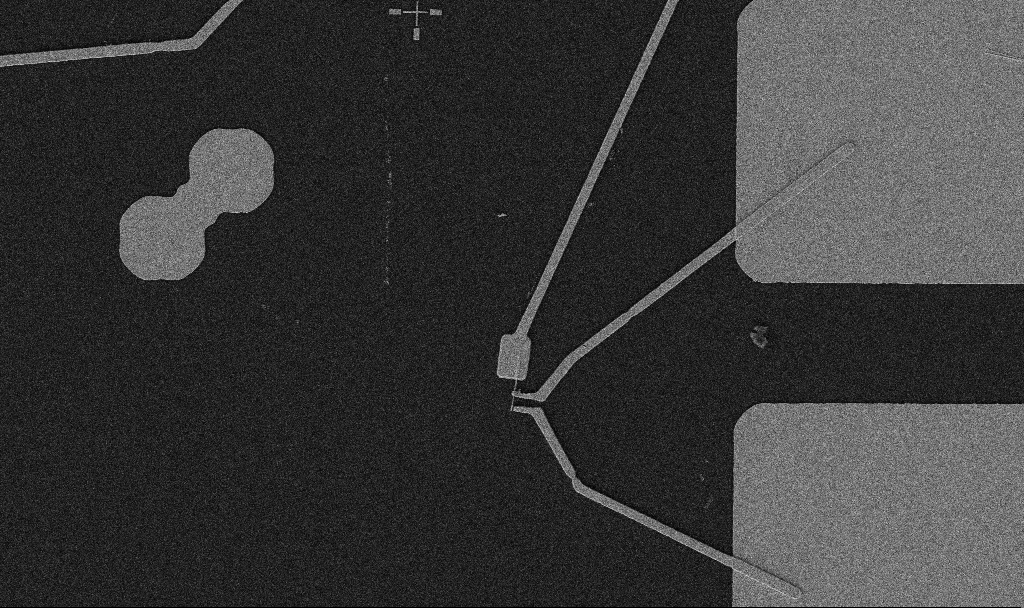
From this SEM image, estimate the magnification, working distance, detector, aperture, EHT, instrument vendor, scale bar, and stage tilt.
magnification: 5 K X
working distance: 10.7 mm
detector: SE2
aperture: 30 µm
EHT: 5 kV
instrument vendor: Zeiss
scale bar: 10000 nm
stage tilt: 0°